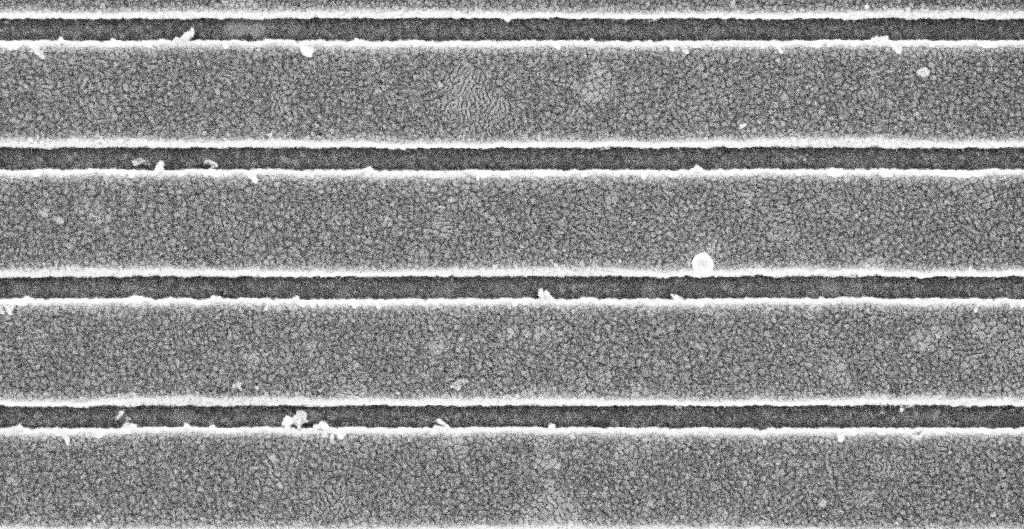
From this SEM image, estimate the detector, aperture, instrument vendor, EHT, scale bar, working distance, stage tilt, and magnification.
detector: InLens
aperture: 30 µm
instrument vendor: Zeiss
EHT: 5 kV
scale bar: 200 nm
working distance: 5.2 mm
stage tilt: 0°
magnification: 79.3 K X